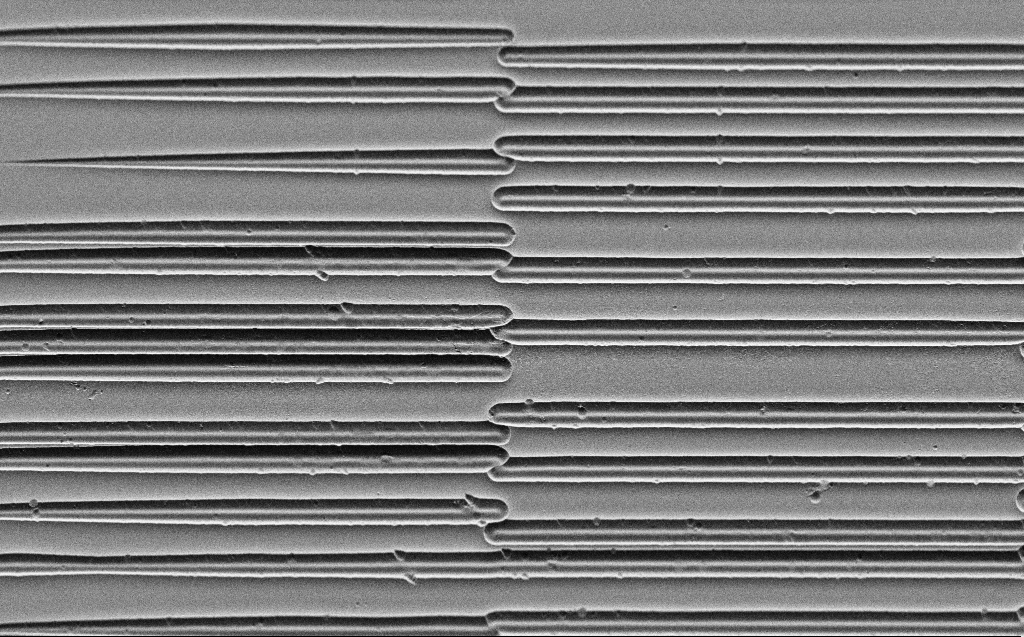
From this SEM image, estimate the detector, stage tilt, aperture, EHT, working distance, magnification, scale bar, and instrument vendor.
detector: SE2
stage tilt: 45°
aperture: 30 µm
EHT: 3 kV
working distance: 6 mm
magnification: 2 K X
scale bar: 10000 nm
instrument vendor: Zeiss